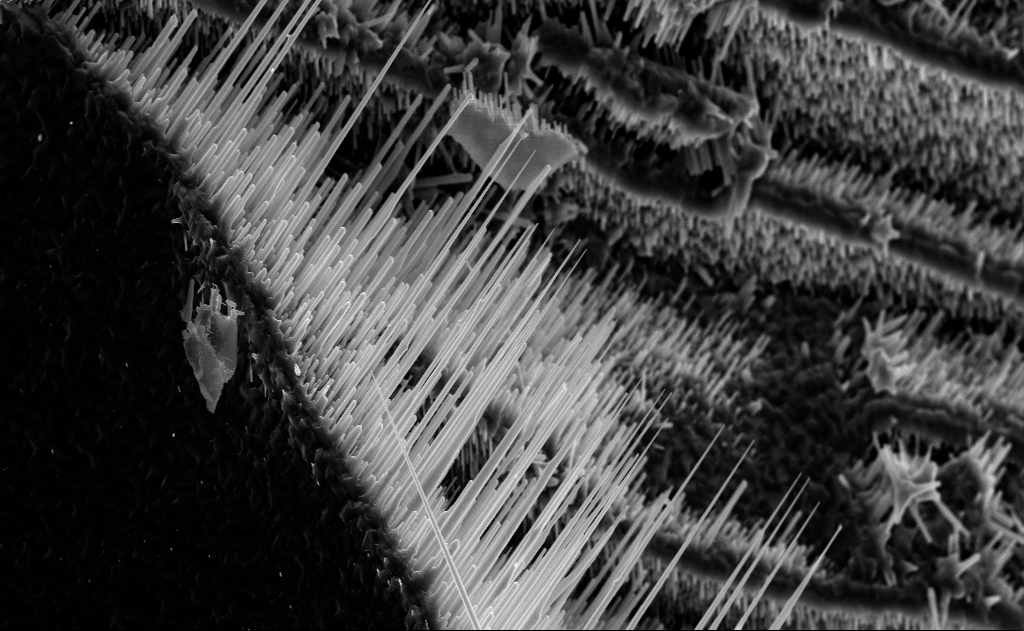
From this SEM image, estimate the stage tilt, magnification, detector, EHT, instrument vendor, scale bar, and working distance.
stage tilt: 0°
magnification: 10 K X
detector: InLens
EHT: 10 kV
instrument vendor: Zeiss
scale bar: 2000 nm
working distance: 6 mm